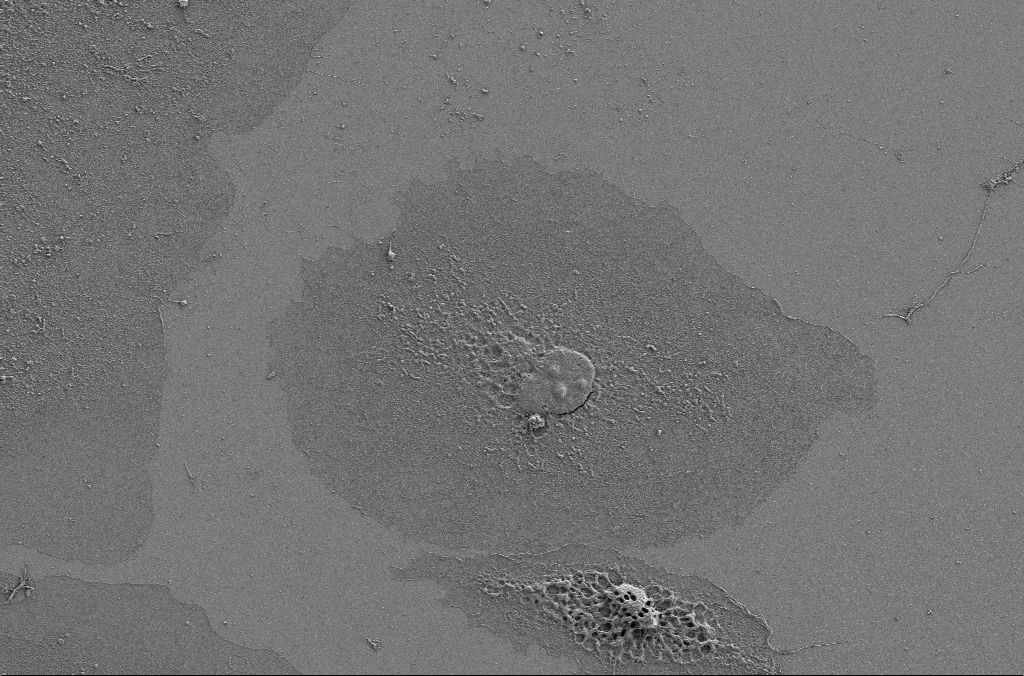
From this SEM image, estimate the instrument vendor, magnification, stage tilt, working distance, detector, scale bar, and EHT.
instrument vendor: Zeiss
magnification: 2.5 K X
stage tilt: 0°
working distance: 4.1 mm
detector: SE2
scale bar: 20000 nm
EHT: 1 kV